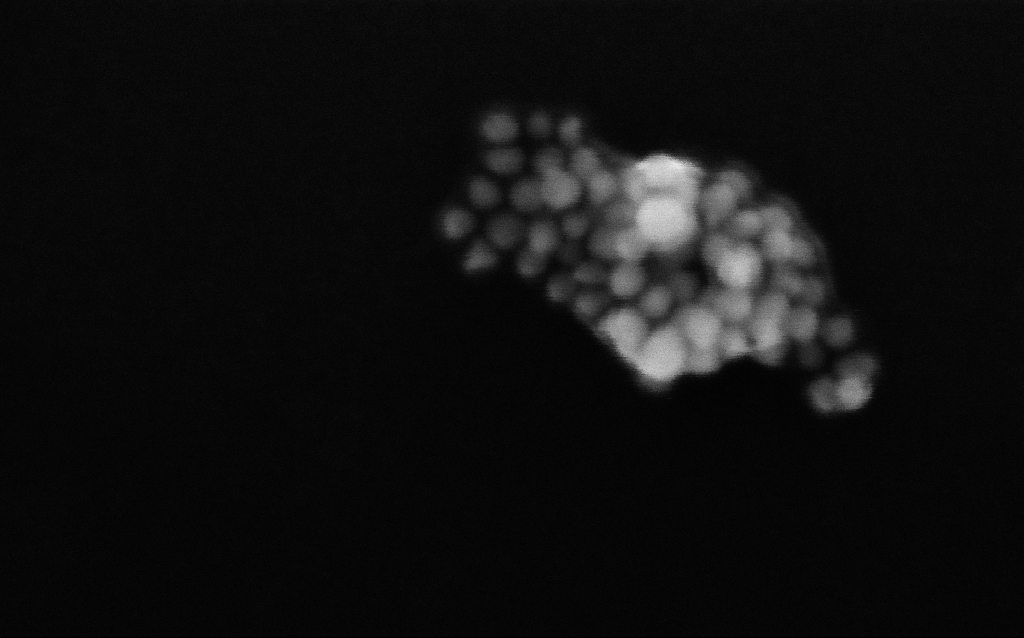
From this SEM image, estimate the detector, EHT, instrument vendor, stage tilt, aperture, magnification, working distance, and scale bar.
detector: InLens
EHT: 6 kV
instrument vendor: Zeiss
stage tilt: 0°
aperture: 30 µm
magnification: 862.85 K X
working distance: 3 mm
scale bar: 20 nm